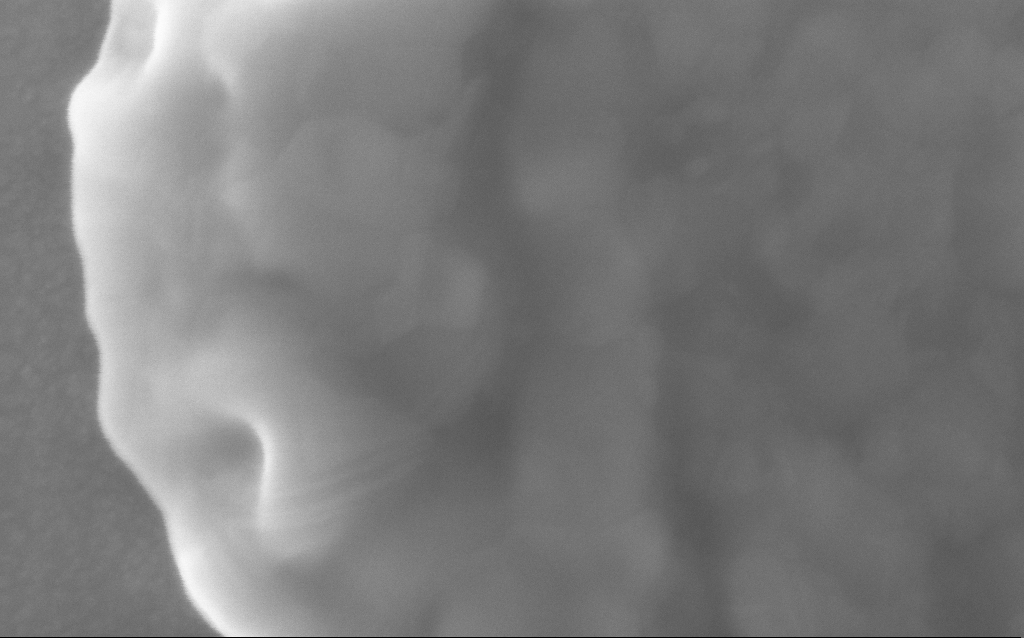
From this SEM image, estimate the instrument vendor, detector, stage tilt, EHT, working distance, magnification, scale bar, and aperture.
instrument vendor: Zeiss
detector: InLens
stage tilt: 0°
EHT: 10 kV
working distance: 2 mm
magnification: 432.22 K X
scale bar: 100 nm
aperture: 30 µm